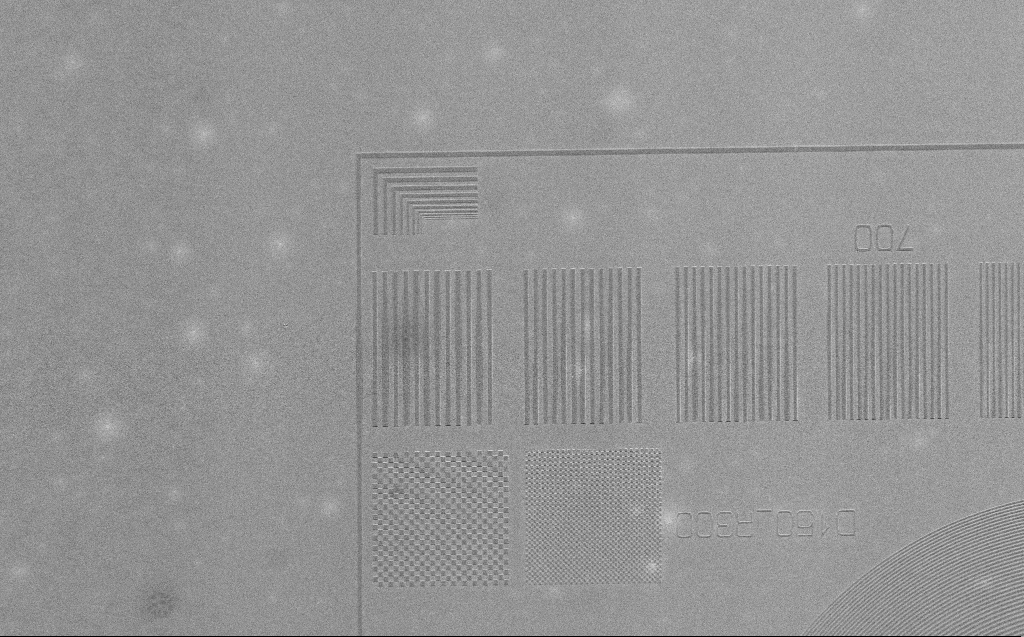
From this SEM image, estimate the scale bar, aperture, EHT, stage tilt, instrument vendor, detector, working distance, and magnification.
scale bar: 10000 nm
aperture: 30 µm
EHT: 2.5 kV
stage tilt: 30°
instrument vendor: Zeiss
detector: SE2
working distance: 5 mm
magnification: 1.94 K X